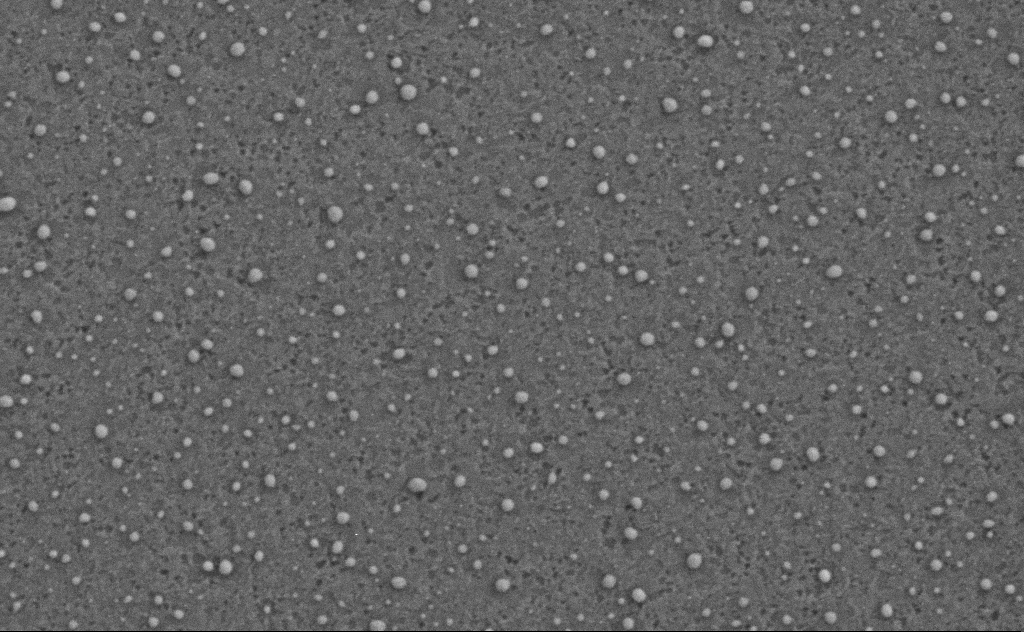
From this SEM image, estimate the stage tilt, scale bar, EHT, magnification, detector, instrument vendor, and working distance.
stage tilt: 0°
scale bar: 200 nm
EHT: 3 kV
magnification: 80 K X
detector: SE2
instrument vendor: Zeiss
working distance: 4 mm